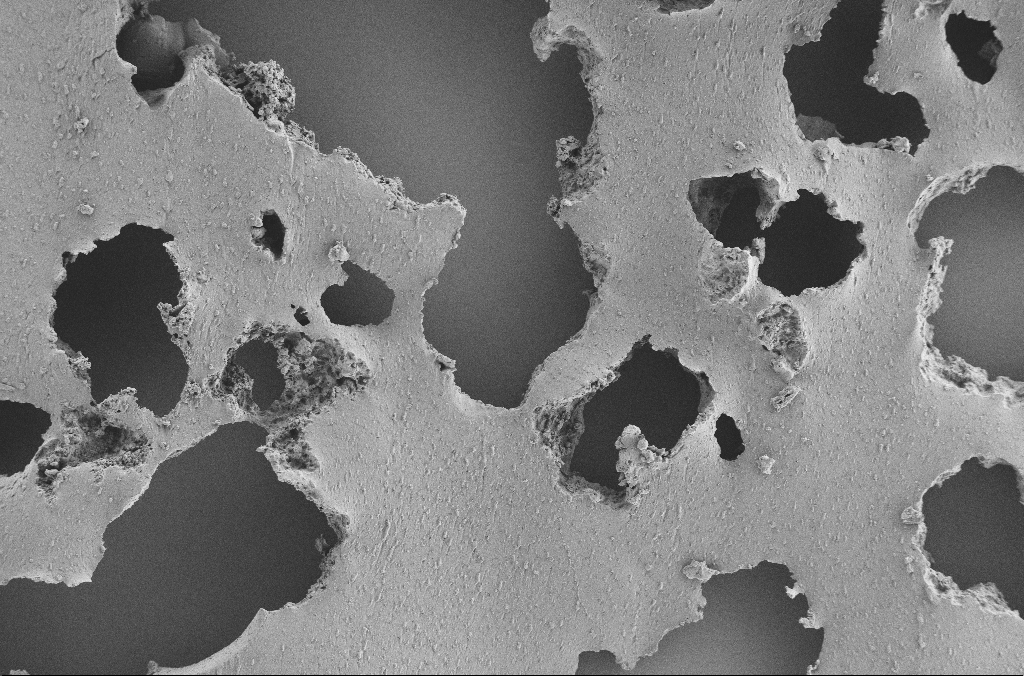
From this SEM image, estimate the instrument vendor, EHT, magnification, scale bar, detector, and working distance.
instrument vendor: Zeiss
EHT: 2 kV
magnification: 0.25 K X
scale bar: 100000 nm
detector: SE2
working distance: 3.6 mm